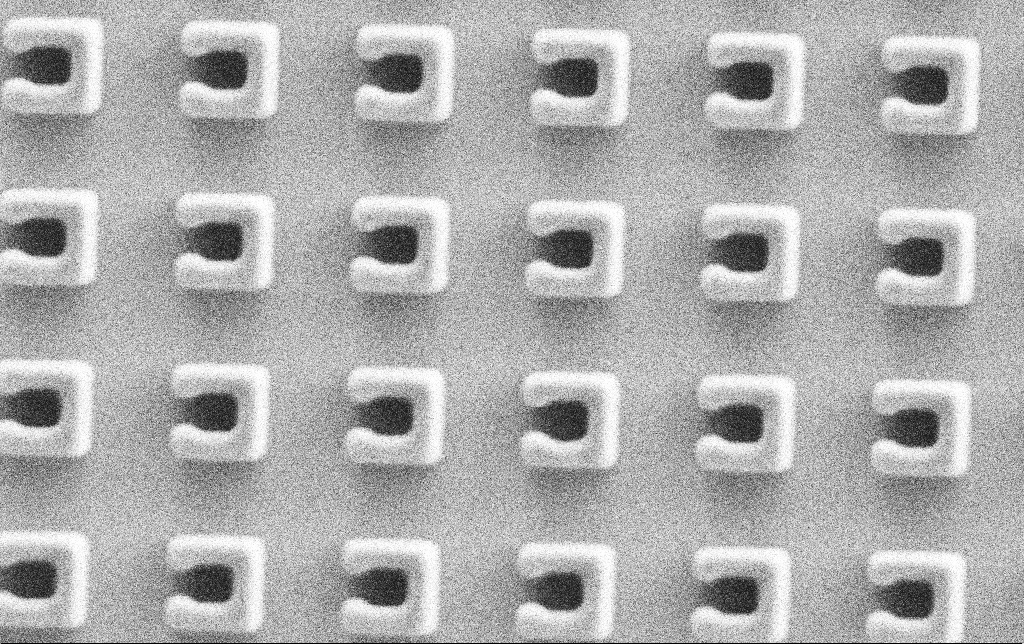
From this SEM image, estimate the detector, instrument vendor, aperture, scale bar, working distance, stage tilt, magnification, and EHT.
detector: SE2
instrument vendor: Zeiss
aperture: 30 µm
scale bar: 1000 nm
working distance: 7.4 mm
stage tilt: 0°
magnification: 64.01 K X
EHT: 1.5 kV